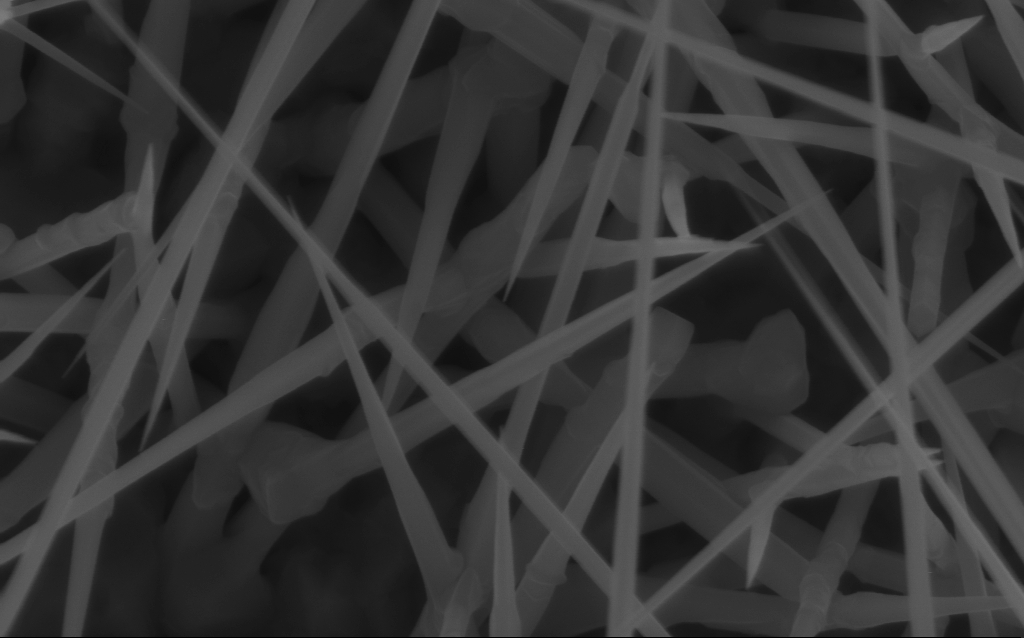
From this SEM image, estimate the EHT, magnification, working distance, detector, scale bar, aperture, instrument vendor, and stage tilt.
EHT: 10 kV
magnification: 80 K X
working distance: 7 mm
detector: InLens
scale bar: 200 nm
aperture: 30 µm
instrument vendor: Zeiss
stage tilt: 0°